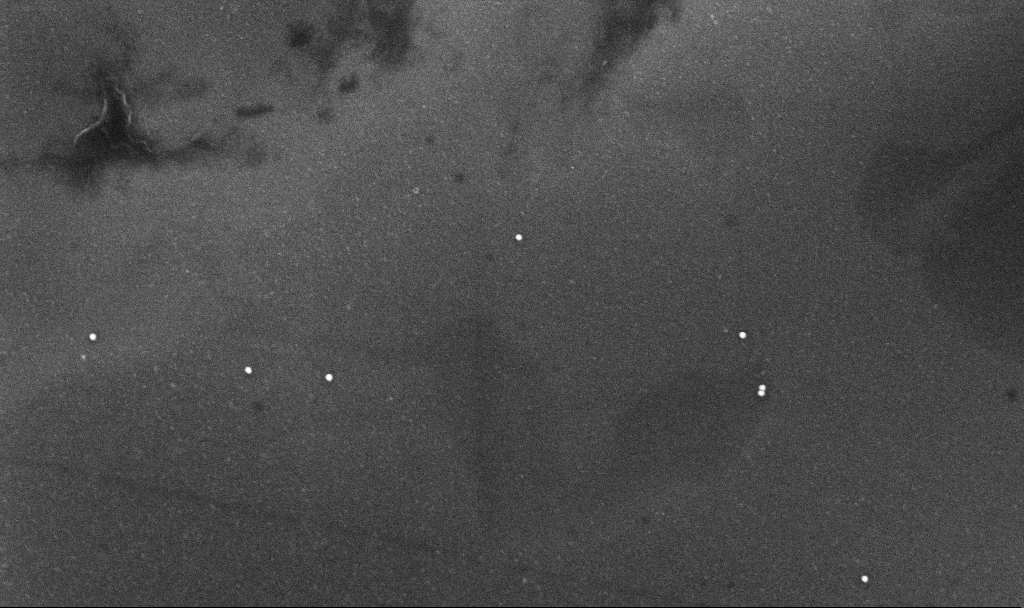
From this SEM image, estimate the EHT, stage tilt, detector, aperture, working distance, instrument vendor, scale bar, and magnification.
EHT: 10 kV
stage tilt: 0°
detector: InLens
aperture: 30 µm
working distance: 3.2 mm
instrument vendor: Zeiss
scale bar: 200 nm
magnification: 80 K X